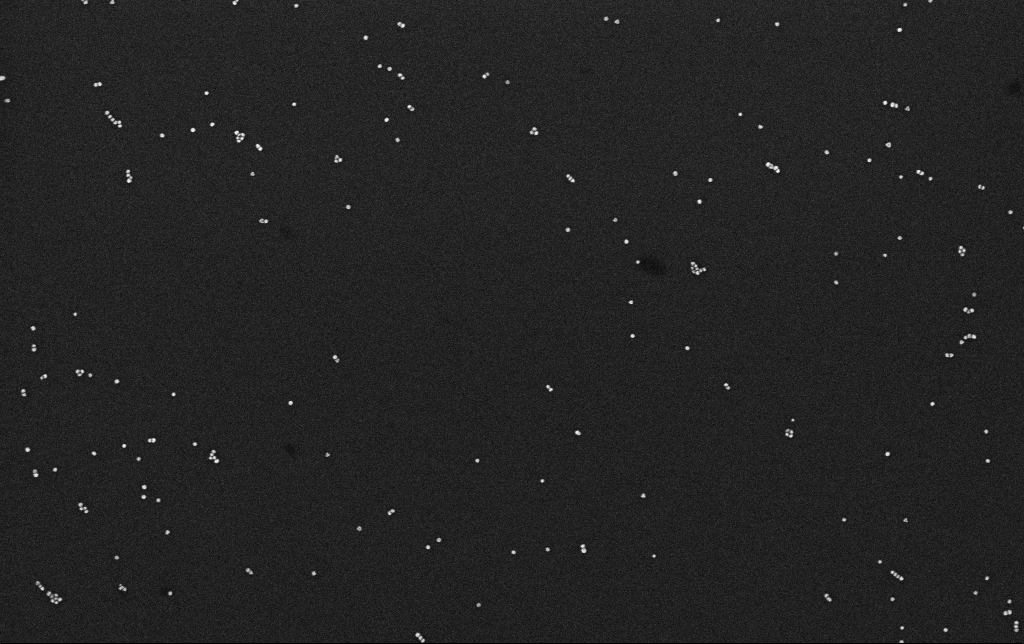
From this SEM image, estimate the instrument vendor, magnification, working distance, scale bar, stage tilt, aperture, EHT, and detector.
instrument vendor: Zeiss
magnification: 100 K X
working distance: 3.1 mm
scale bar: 200 nm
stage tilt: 0°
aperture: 30 µm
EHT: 10 kV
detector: InLens